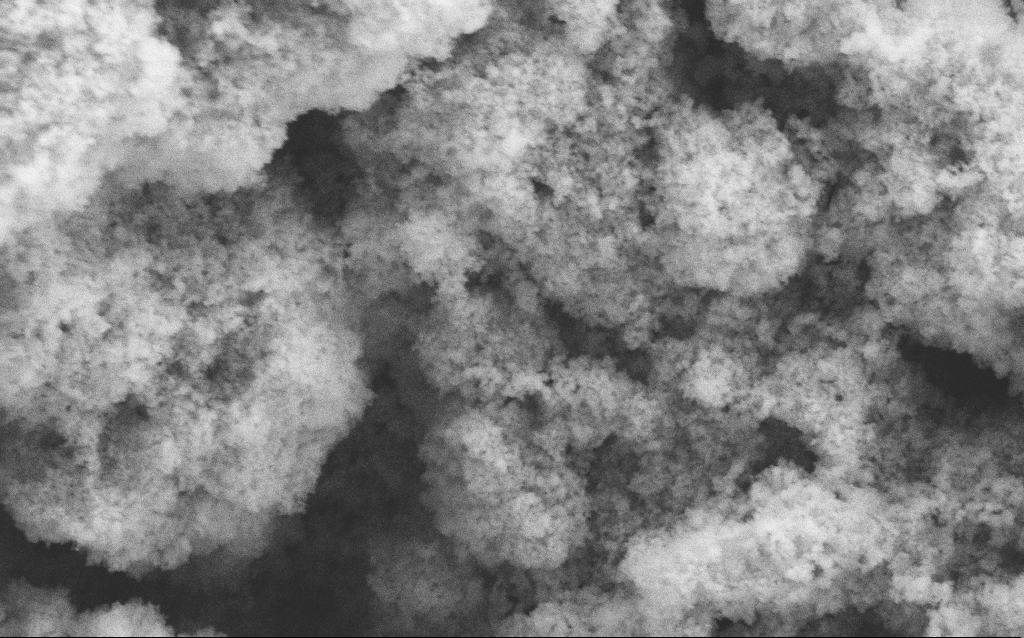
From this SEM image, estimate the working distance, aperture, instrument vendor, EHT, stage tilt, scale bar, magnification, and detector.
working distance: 4.7 mm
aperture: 30 µm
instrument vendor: Zeiss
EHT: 5 kV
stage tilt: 0°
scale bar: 1000 nm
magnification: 68.64 K X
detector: SE2